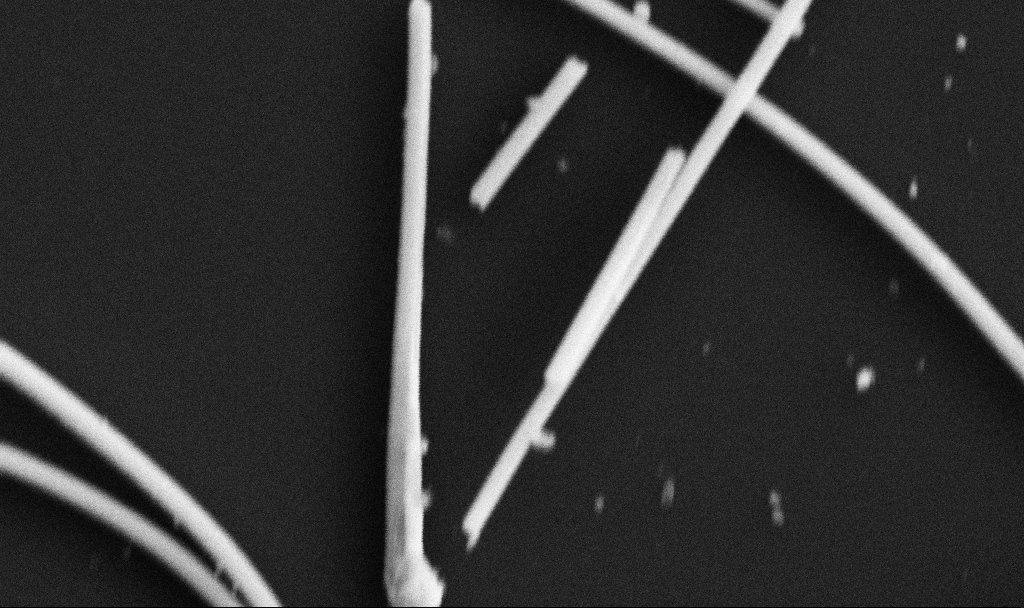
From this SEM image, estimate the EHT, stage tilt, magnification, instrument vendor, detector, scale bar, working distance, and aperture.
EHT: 5 kV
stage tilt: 0°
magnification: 84.74 K X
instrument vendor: Zeiss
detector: SE2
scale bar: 200 nm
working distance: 10.7 mm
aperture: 30 µm